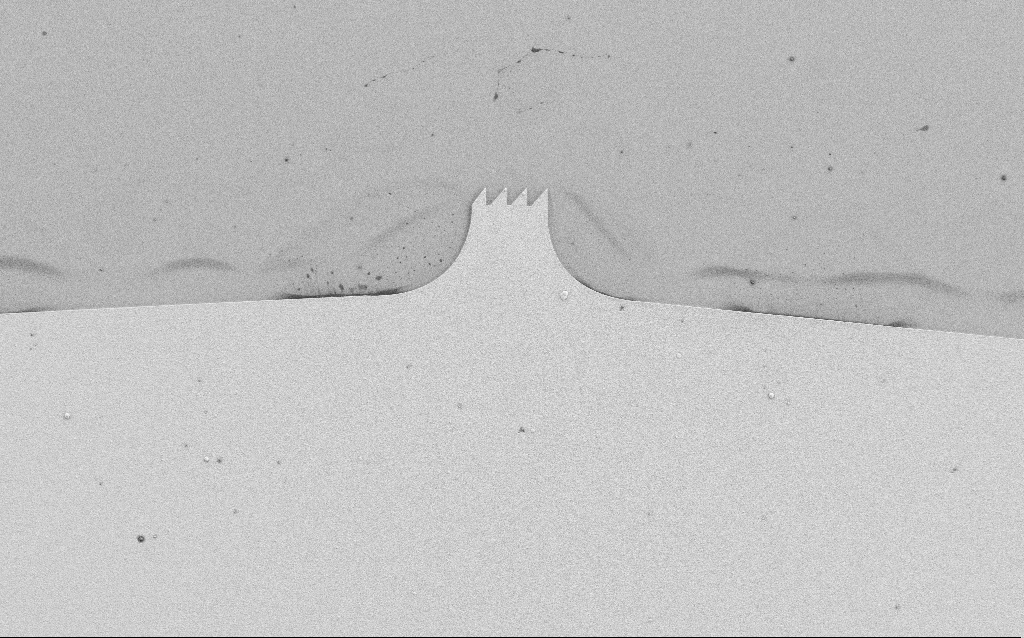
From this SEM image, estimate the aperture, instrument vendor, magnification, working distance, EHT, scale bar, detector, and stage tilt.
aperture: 30 µm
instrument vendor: Zeiss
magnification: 0.665 K X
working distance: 4.5 mm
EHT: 3 kV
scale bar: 100000 nm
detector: SE2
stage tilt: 0°